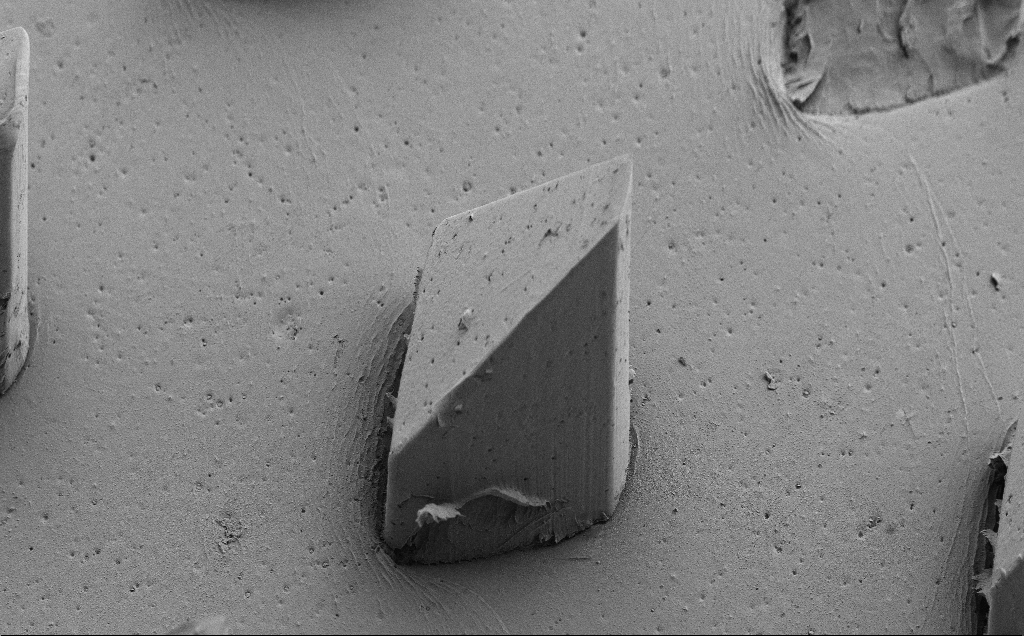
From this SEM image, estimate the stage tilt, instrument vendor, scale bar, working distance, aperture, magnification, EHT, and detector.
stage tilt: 39°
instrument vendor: Zeiss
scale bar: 200000 nm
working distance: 8 mm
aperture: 30 µm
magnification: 0.235 K X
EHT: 5 kV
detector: SE2